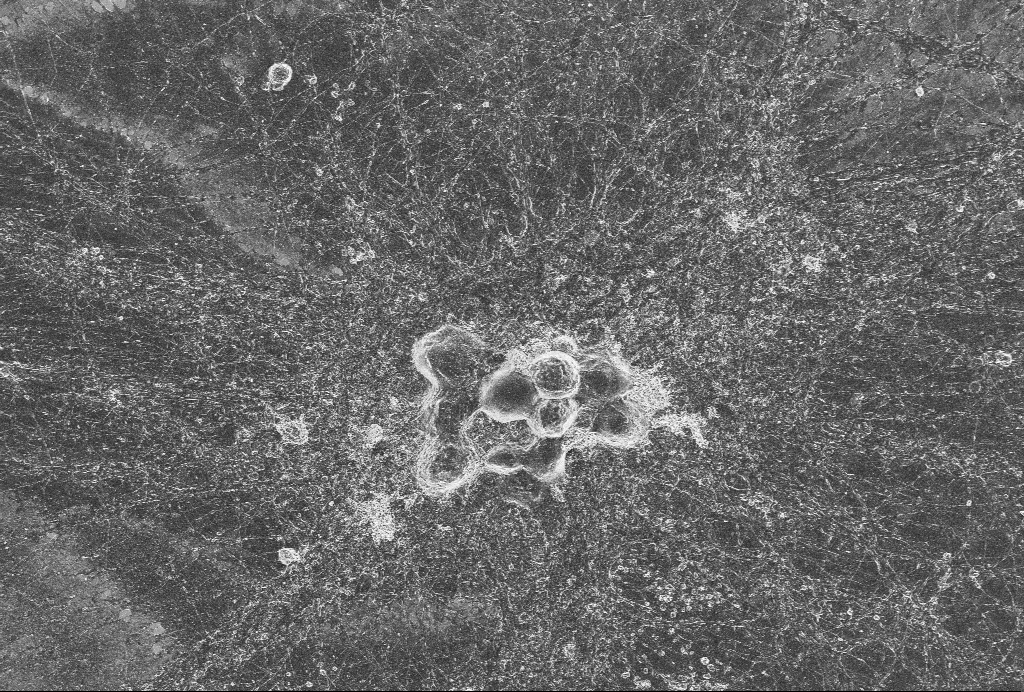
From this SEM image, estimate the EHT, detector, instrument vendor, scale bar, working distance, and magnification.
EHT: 2 kV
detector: InLens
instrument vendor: Zeiss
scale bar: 20000 nm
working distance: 6 mm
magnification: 2 K X